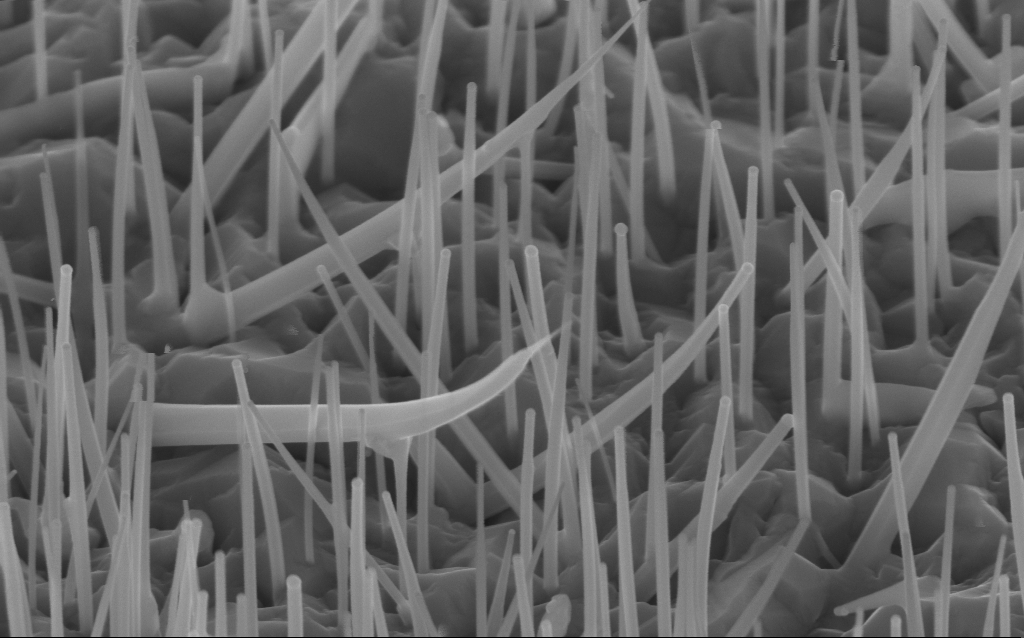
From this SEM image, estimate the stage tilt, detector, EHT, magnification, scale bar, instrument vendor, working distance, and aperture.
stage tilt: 45°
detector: InLens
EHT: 10 kV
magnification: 82.53 K X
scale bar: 200 nm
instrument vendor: Zeiss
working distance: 6 mm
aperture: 30 µm